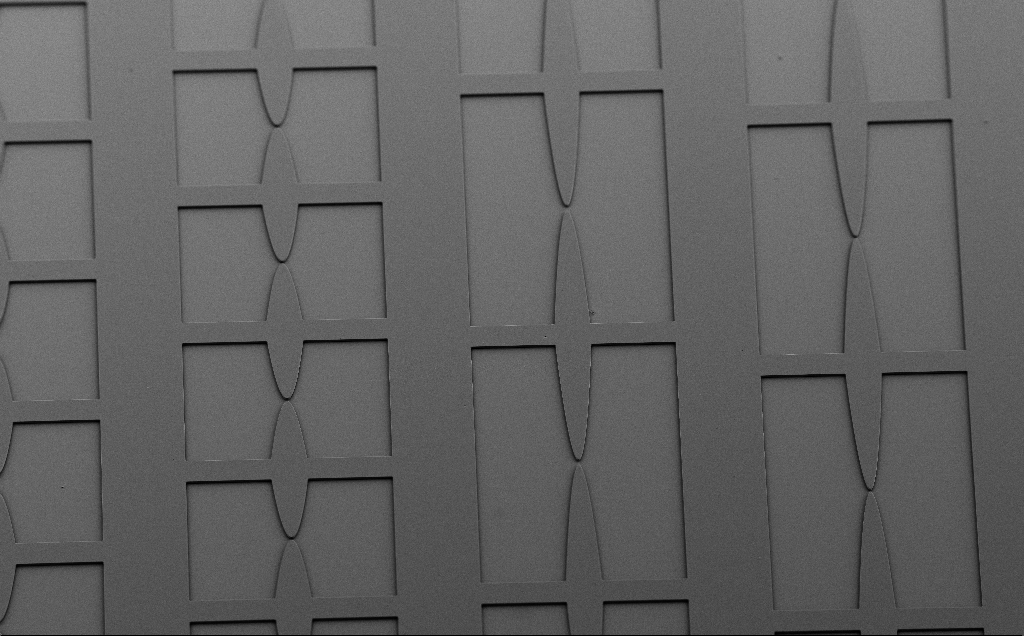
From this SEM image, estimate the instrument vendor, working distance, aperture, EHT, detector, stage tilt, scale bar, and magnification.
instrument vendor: Zeiss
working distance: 9 mm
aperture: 30 µm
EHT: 5 kV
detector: SE2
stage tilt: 40°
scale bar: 200000 nm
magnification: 0.207 K X